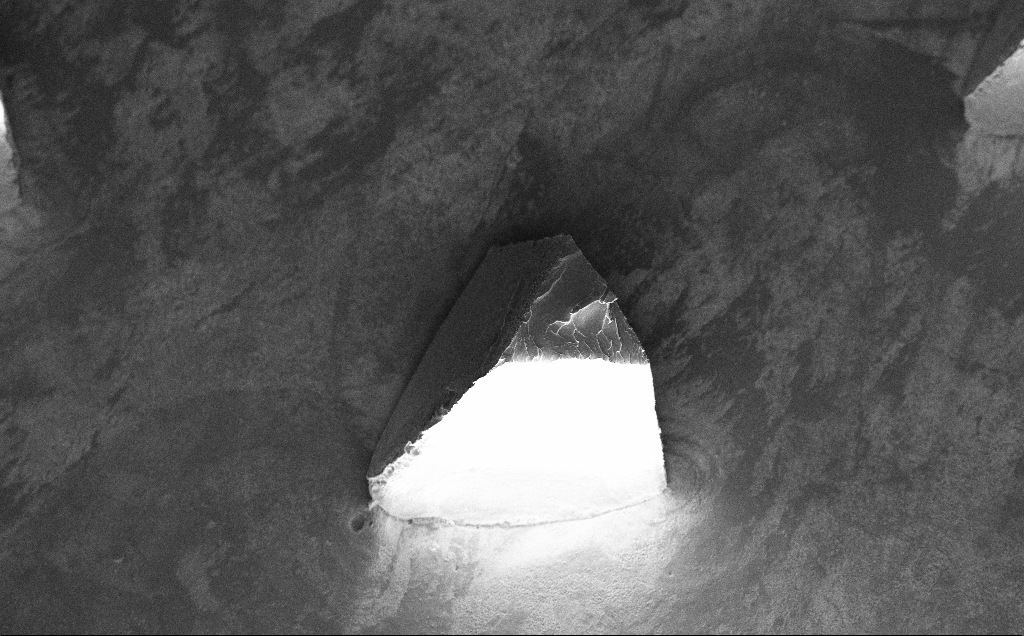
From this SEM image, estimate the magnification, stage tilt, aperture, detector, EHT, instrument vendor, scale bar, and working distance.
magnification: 0.189 K X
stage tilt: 30°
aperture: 30 µm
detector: InLens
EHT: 10 kV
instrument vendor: Zeiss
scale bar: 100000 nm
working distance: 9 mm